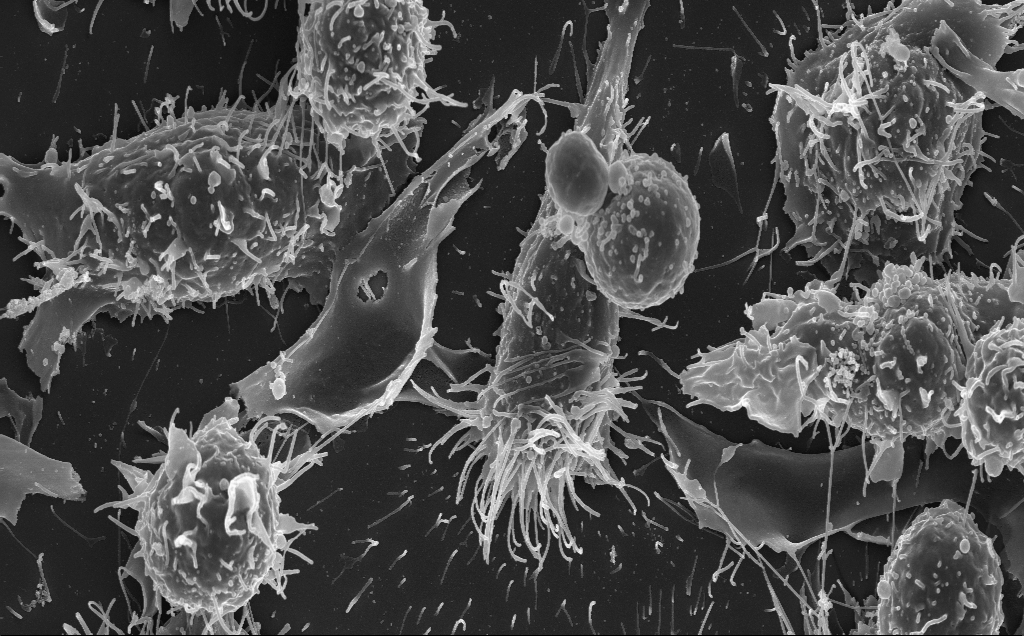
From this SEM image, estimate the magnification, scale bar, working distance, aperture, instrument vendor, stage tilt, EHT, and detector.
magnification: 6.29 K X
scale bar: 10000 nm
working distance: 5 mm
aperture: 30 µm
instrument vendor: Zeiss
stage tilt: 0.7°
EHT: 10 kV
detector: InLens